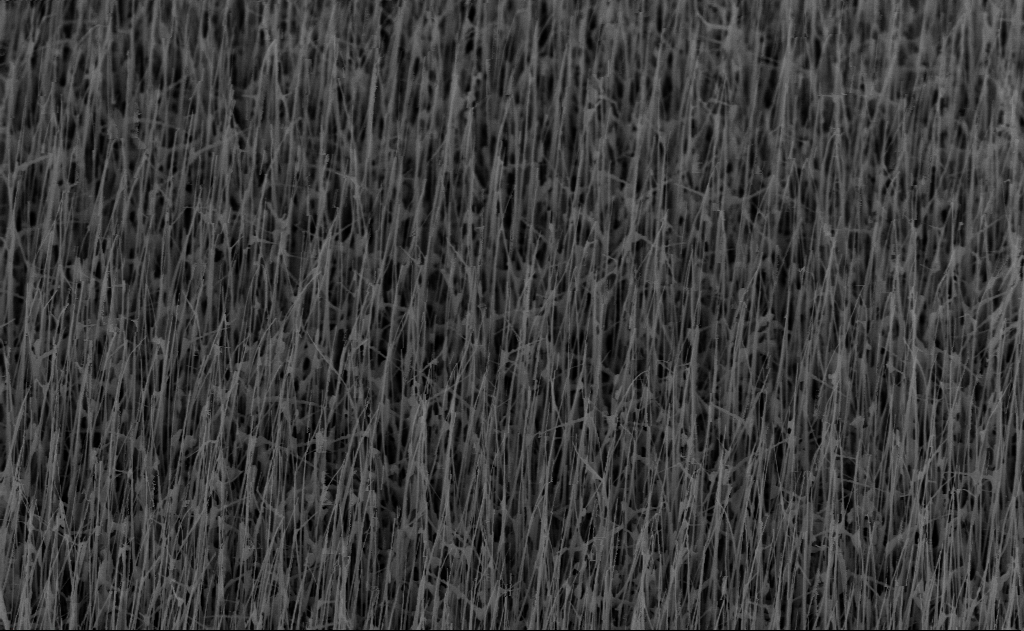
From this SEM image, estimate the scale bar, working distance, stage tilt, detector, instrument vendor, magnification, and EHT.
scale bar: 2000 nm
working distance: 7 mm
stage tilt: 45°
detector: InLens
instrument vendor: Zeiss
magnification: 20 K X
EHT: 10 kV